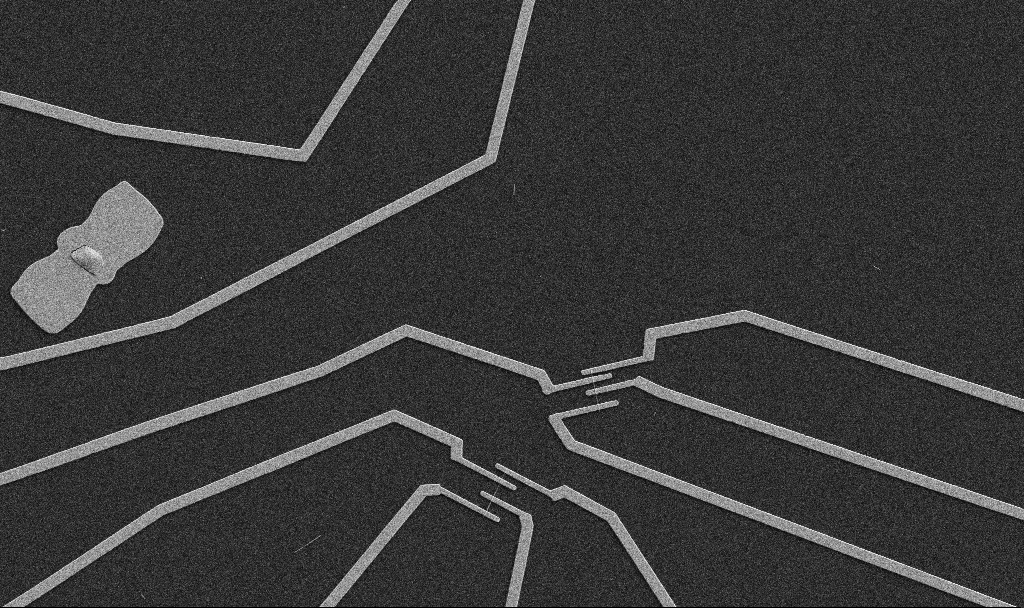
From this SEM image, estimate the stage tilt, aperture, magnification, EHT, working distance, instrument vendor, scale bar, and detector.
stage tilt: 0°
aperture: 30 µm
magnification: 5 K X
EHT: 5 kV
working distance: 10.7 mm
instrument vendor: Zeiss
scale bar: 10000 nm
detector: SE2